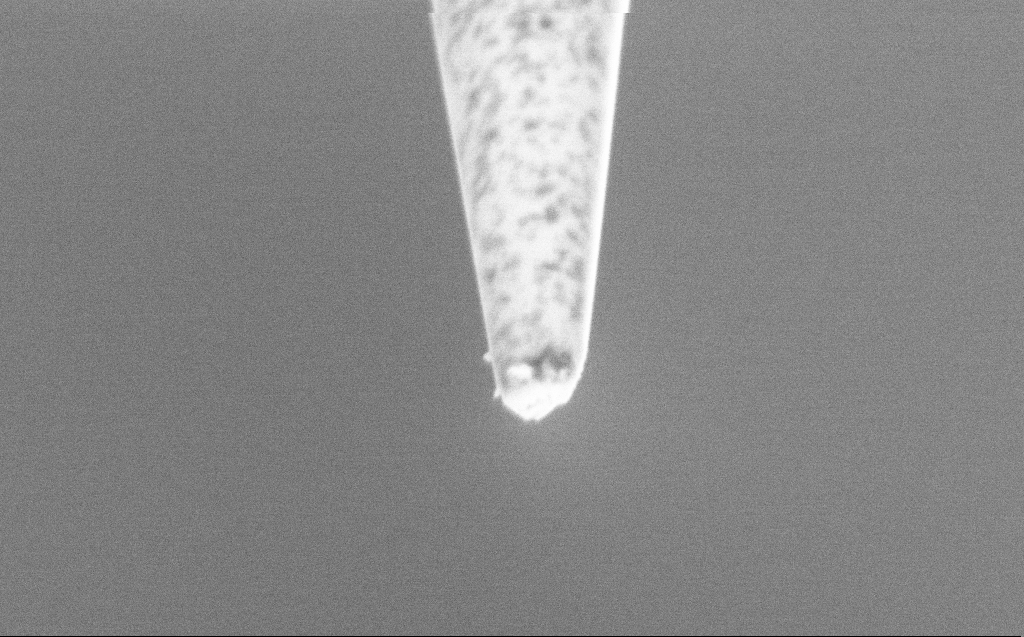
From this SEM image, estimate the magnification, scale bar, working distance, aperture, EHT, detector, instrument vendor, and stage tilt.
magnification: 100 K X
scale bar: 200 nm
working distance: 6 mm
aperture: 30 µm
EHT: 2 kV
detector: SE2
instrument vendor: Zeiss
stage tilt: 45°